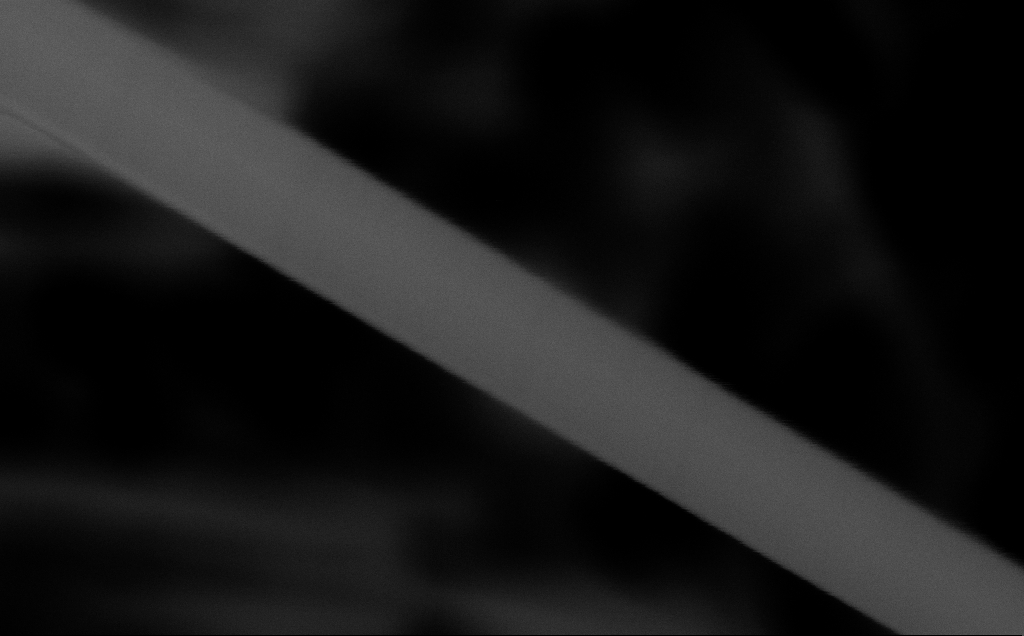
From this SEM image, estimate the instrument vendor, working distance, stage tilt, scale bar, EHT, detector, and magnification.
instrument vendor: Zeiss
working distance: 6 mm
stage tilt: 0°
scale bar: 100 nm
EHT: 10 kV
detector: InLens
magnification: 437.62 K X